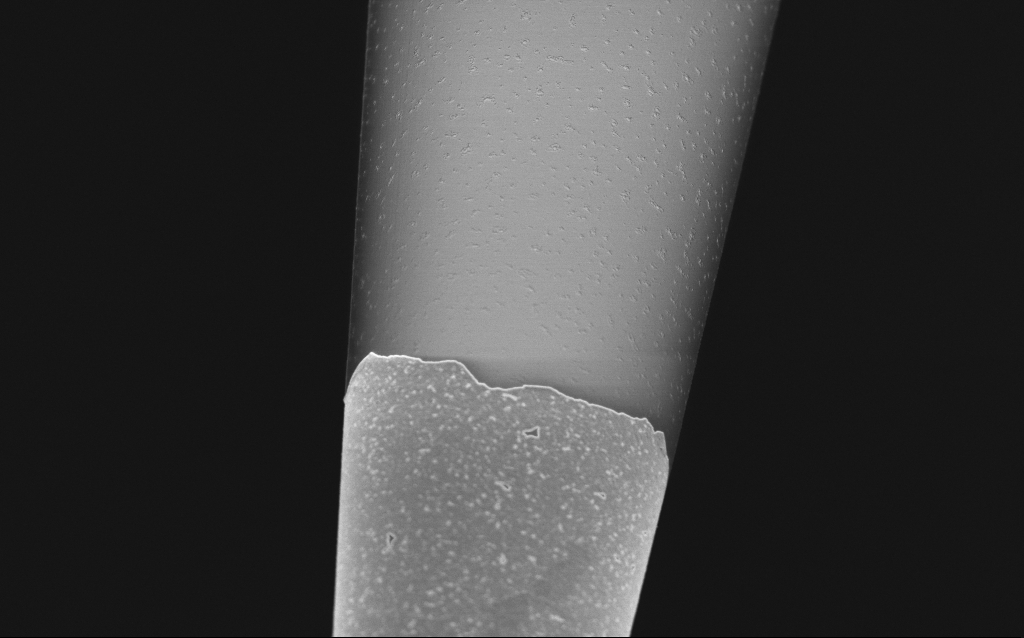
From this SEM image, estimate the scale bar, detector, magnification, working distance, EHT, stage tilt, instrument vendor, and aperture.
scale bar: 2000 nm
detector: InLens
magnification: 10 K X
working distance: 6 mm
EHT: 1 kV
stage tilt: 45°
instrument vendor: Zeiss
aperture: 30 µm